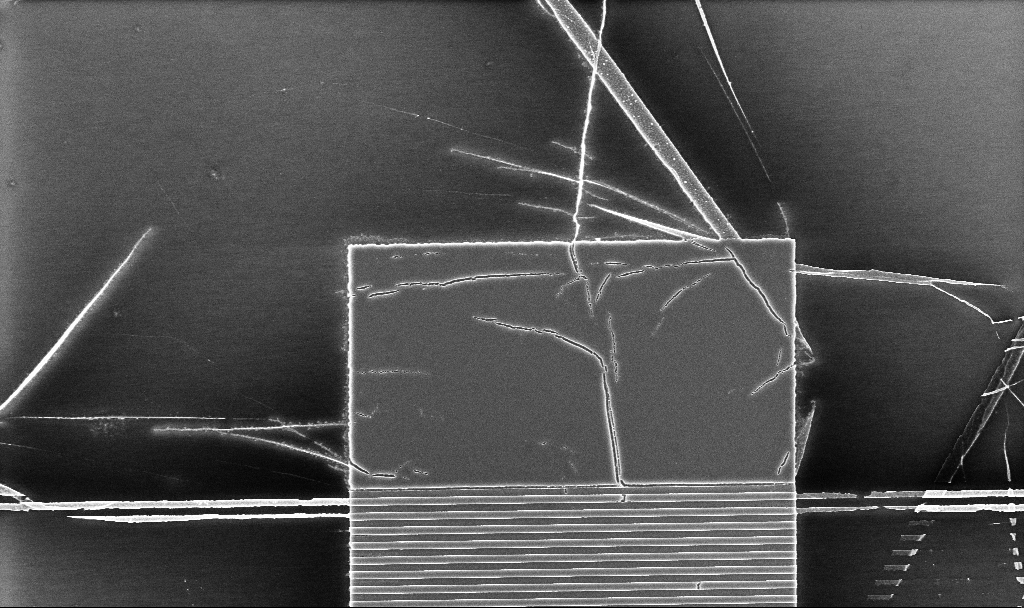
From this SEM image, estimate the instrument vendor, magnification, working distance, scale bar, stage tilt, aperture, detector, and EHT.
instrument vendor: Zeiss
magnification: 8.33 K X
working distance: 5.2 mm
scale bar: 2000 nm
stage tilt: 0°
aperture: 30 µm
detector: InLens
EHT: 5 kV